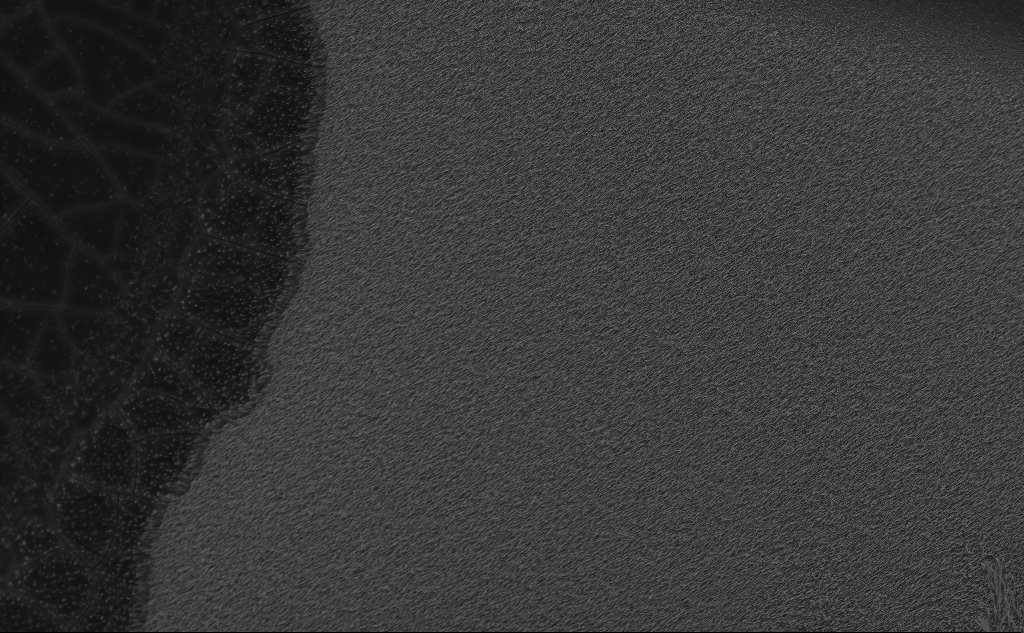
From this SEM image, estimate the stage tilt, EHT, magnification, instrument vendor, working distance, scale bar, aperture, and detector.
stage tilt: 45°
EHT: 10 kV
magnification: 2.96 K X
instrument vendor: Zeiss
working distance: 6 mm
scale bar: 10000 nm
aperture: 30 µm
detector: InLens